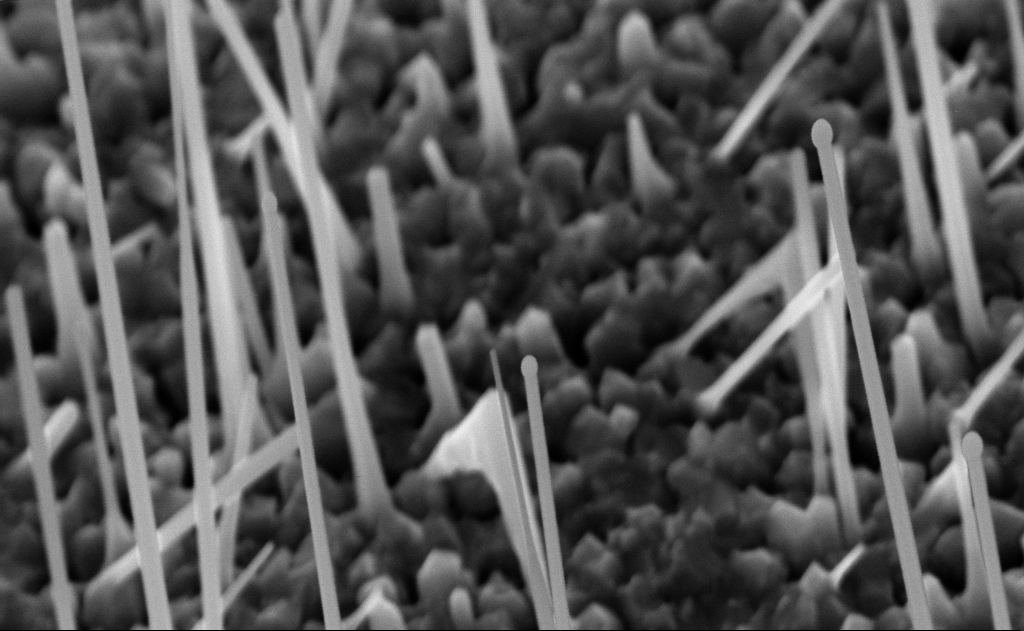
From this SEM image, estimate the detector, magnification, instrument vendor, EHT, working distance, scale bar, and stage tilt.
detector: InLens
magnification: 80 K X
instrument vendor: Zeiss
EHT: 10 kV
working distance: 8 mm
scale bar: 200 nm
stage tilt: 0°